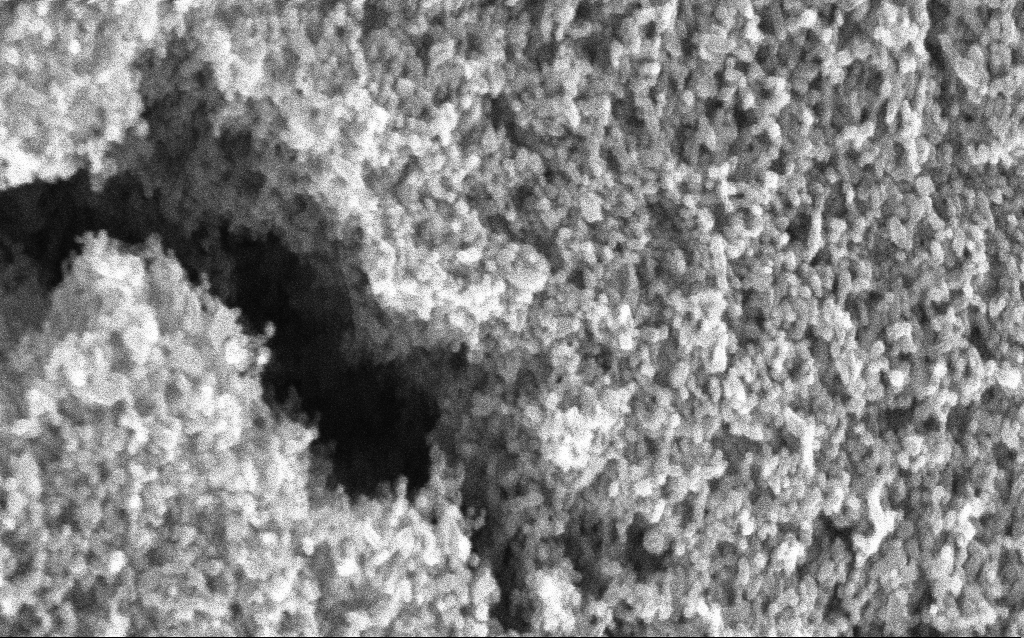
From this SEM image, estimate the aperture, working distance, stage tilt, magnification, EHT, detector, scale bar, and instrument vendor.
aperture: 30 µm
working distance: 2.6 mm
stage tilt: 0°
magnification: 204.13 K X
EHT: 5 kV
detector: InLens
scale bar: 100 nm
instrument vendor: Zeiss